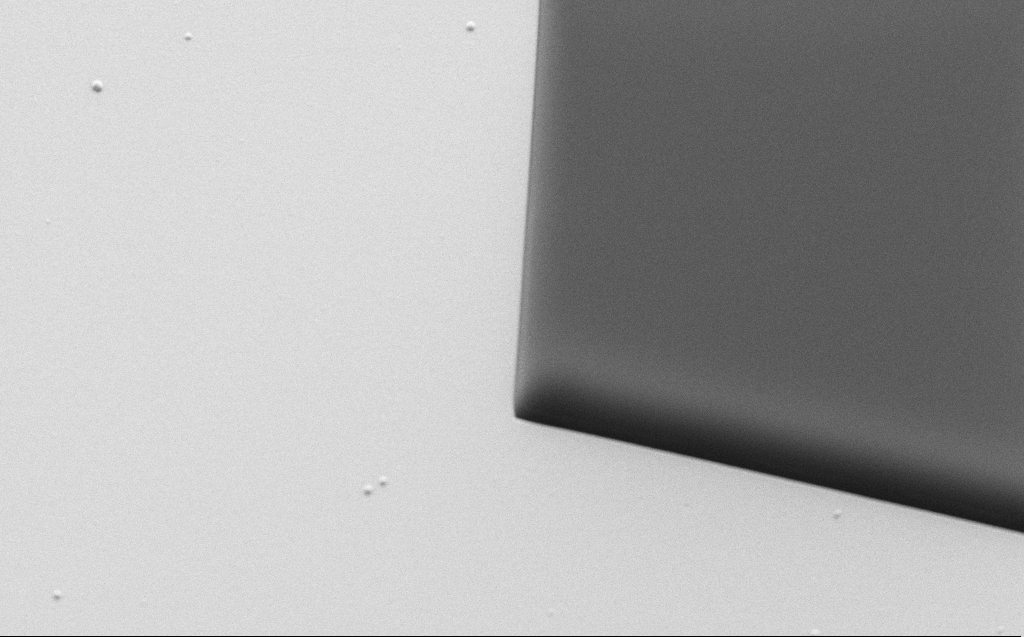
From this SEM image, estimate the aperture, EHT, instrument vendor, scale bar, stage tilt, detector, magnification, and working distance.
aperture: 30 µm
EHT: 1.1 kV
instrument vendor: Zeiss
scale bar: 20000 nm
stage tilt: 30°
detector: SE2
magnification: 2.64 K X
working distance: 6 mm